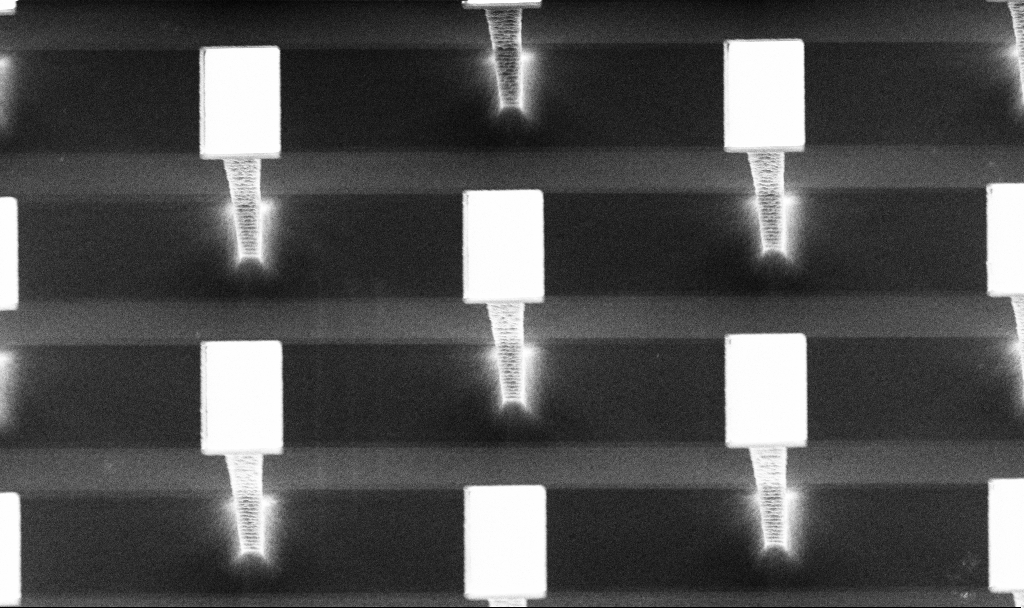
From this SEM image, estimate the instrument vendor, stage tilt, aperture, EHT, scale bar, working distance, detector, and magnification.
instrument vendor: Zeiss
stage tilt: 20°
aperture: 30 µm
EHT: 5 kV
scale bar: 2000 nm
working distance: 4.2 mm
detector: InLens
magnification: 9.7 K X